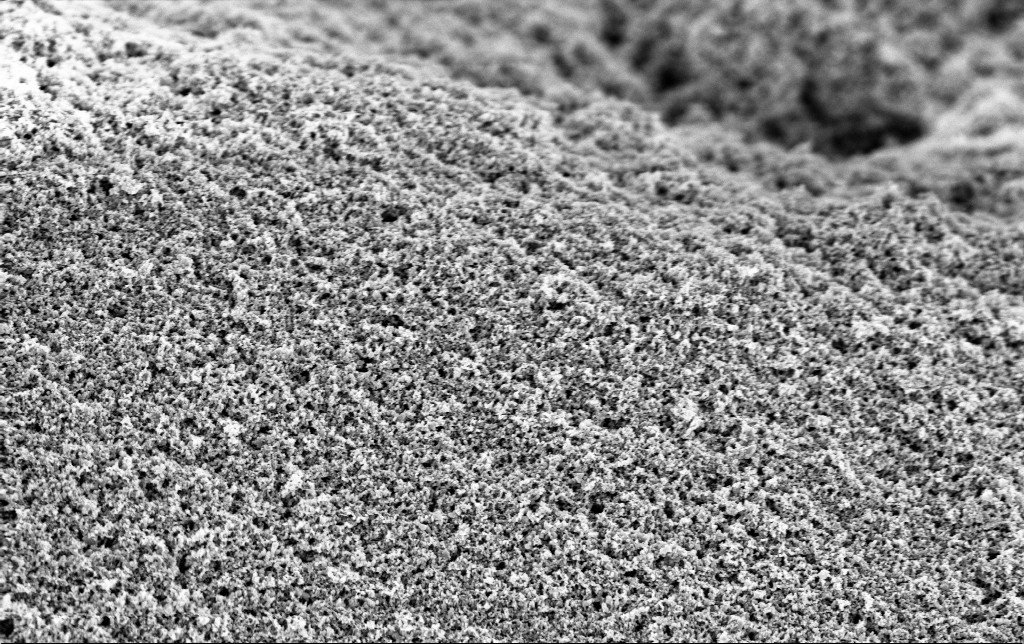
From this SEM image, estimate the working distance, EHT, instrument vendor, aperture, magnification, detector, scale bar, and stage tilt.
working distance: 2.8 mm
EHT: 3 kV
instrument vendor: Zeiss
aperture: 30 µm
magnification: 30 K X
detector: InLens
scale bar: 2000 nm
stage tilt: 0°